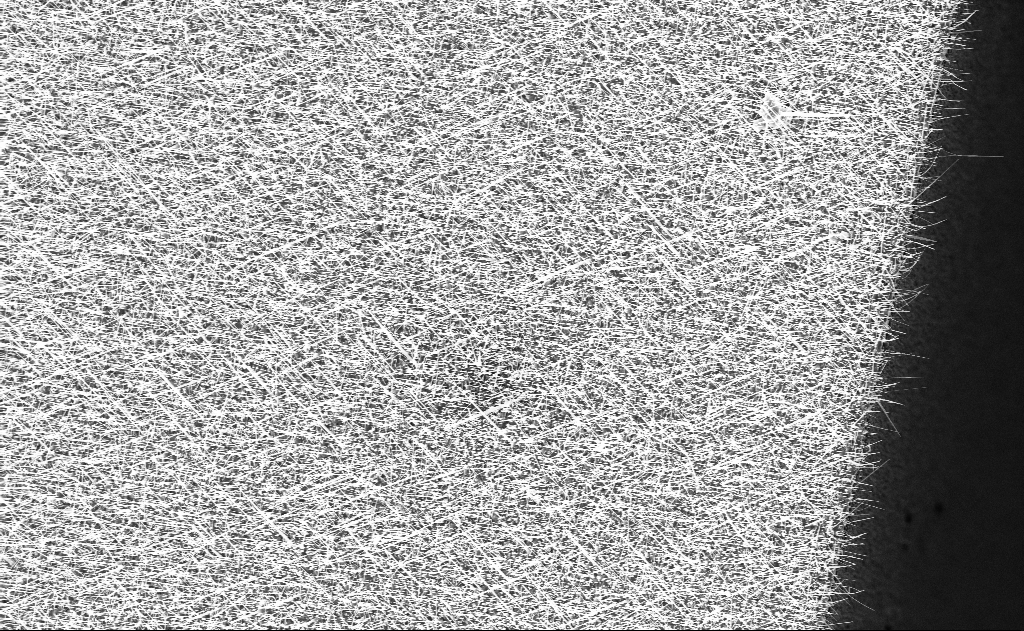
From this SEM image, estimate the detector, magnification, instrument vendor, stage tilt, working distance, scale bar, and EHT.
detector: InLens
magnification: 5 K X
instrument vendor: Zeiss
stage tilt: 0°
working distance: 10 mm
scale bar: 10000 nm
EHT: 10 kV